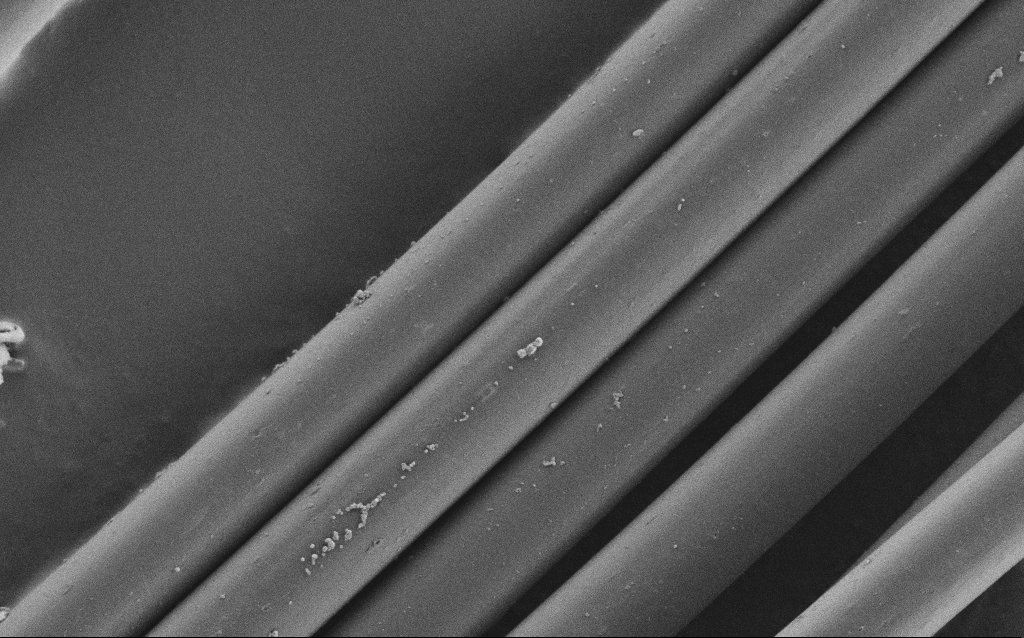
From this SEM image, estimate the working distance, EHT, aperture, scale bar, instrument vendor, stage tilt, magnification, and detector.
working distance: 5 mm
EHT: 1 kV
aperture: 30 µm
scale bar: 20000 nm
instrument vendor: Zeiss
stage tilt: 0°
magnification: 2.97 K X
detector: SE2